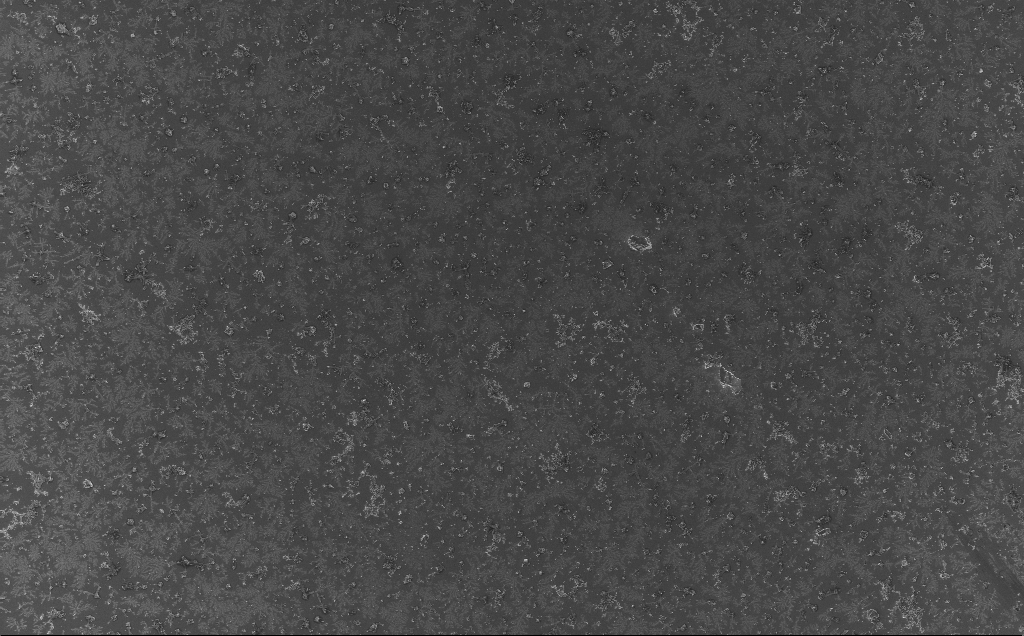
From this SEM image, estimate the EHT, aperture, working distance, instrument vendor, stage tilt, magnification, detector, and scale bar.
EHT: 5 kV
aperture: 30 µm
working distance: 3 mm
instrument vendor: Zeiss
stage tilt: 0°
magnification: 0.959 K X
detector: InLens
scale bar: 20000 nm